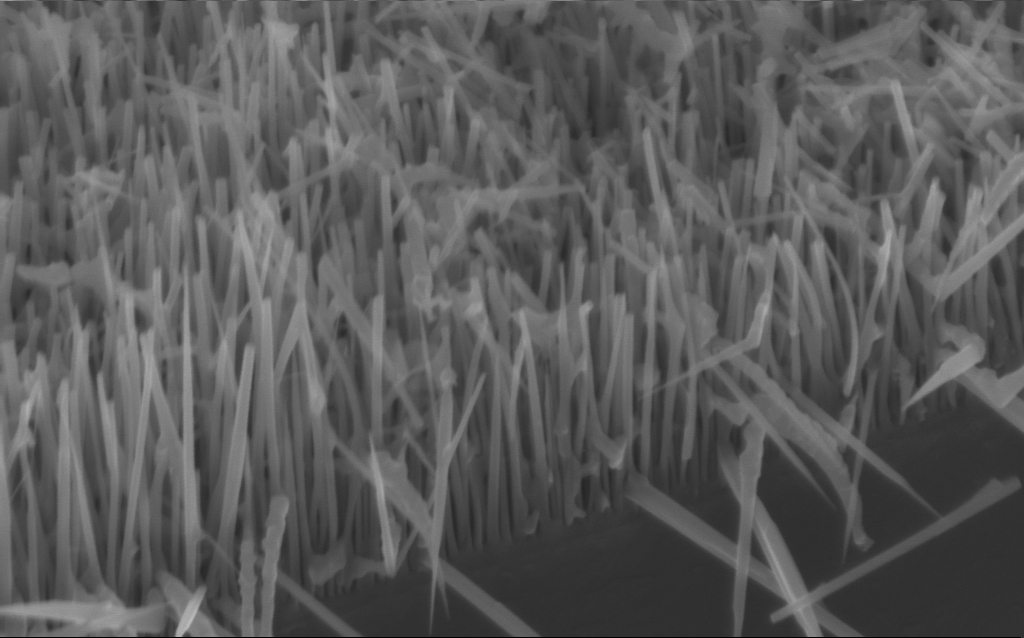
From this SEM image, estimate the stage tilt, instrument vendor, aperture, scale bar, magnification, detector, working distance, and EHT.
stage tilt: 45°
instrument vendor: Zeiss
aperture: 30 µm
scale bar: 1000 nm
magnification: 70.48 K X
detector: InLens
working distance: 6 mm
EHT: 10 kV